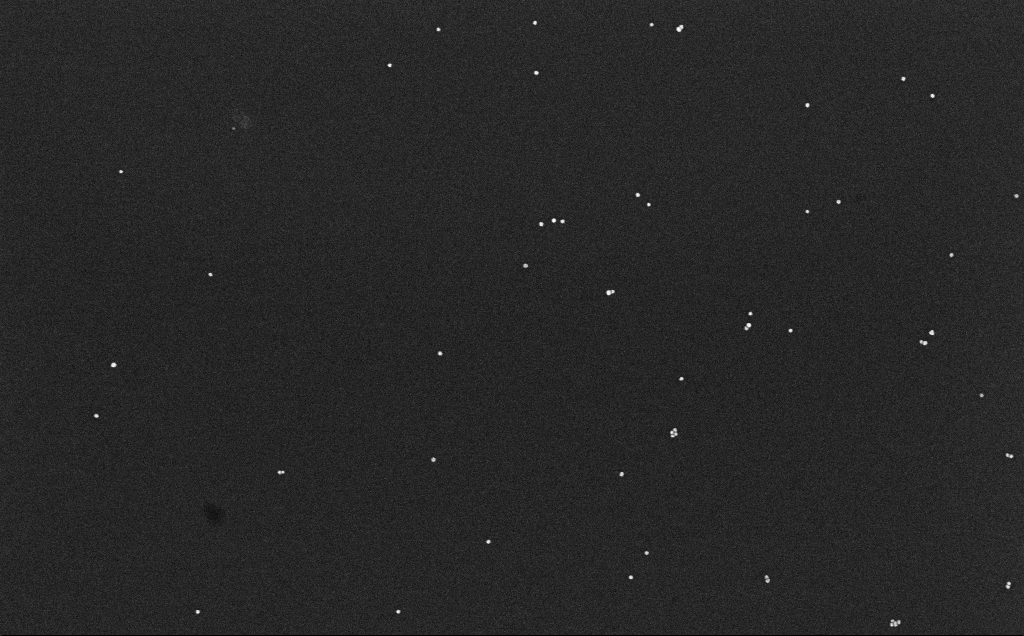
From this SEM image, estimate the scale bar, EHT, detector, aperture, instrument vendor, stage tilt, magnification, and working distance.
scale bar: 200 nm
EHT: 10 kV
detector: InLens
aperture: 30 µm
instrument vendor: Zeiss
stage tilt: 0°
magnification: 100 K X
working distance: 6.6 mm